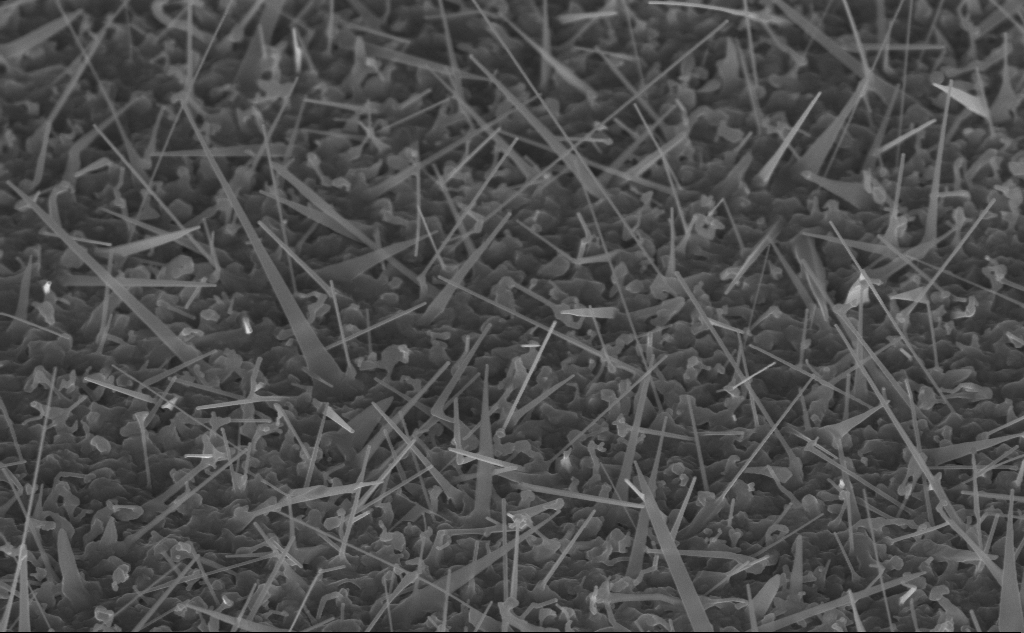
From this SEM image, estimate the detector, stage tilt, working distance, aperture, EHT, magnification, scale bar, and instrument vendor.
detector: InLens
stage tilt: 45°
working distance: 8 mm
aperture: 30 µm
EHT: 10 kV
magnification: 40 K X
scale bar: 1000 nm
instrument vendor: Zeiss